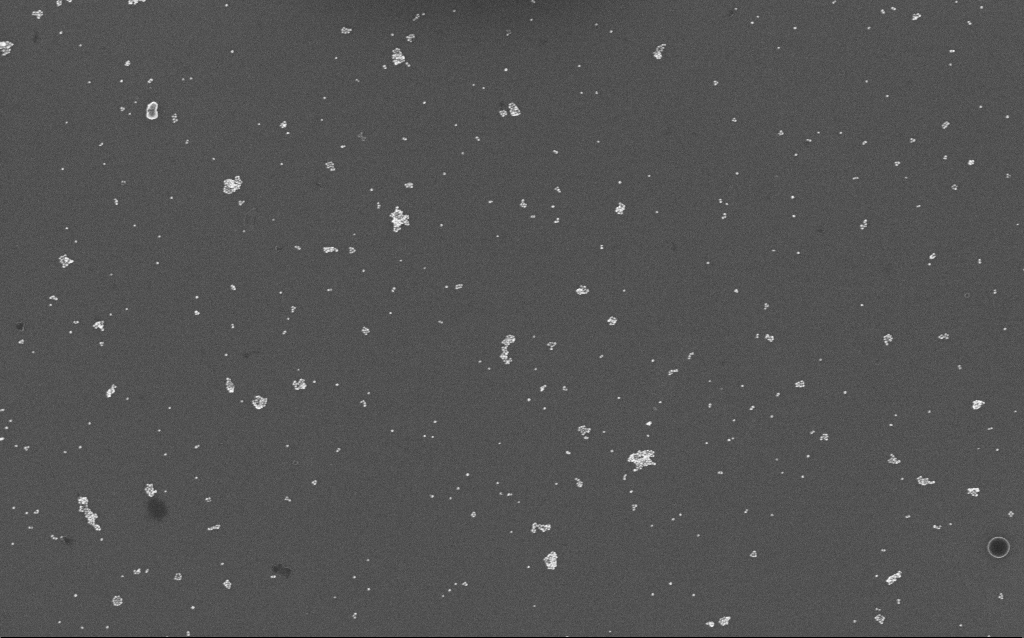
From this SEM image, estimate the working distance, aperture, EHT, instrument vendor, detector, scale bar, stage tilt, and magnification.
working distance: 7 mm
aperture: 30 µm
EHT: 10 kV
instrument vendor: Zeiss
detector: InLens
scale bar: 1000 nm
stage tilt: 0°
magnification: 48.51 K X